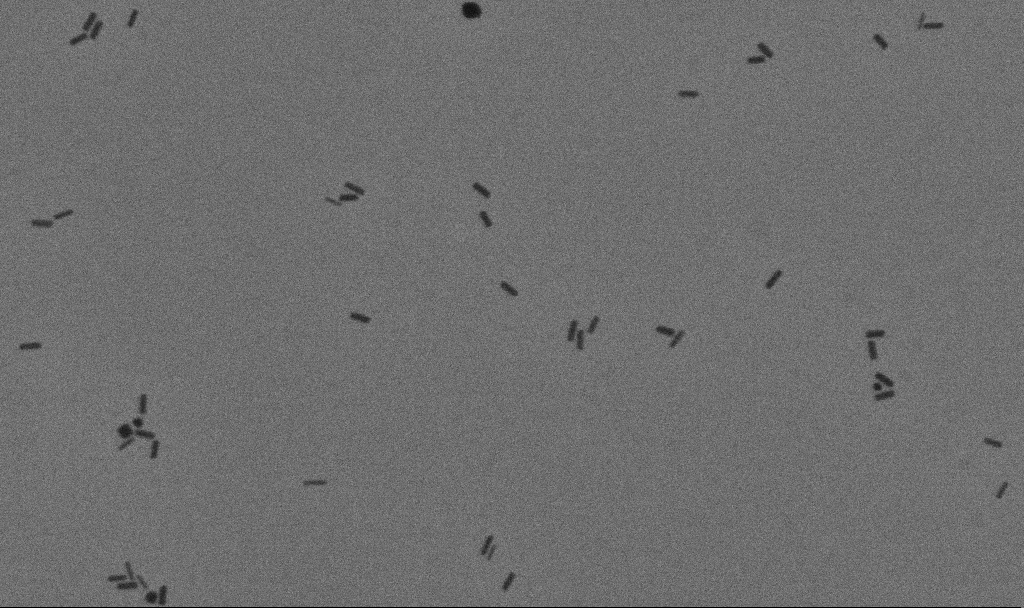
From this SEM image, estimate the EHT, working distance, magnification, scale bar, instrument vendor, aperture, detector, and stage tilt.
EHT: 10 kV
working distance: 11.3 mm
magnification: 100 K X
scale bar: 200 nm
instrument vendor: Zeiss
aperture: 30 µm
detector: SE2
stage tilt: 0°